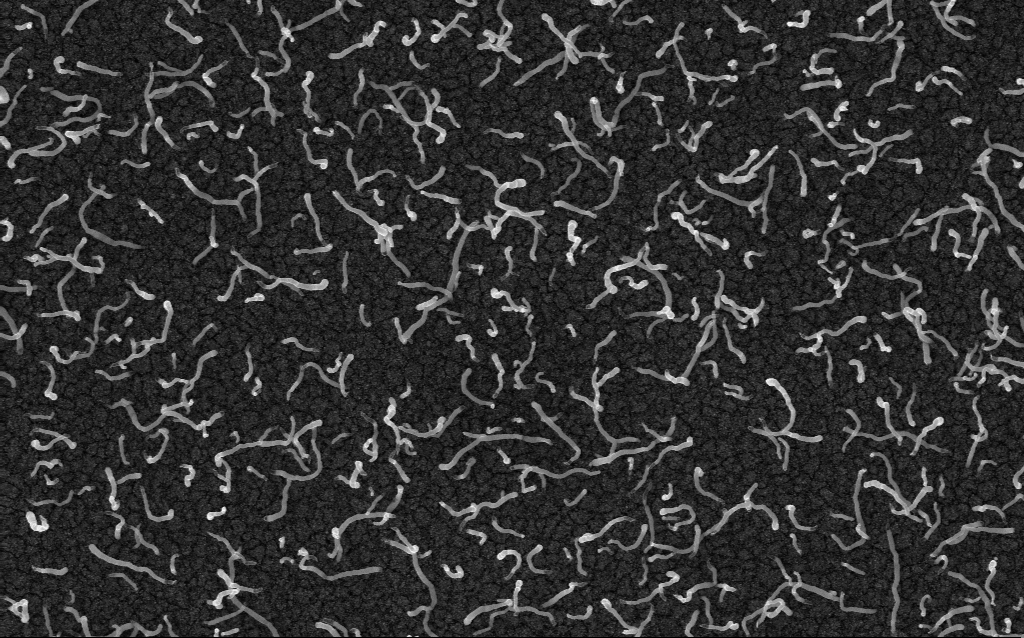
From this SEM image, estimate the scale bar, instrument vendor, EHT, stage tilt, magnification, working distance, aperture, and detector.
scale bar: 1000 nm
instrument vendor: Zeiss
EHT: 5 kV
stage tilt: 0°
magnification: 50 K X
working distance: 2 mm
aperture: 30 µm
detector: InLens